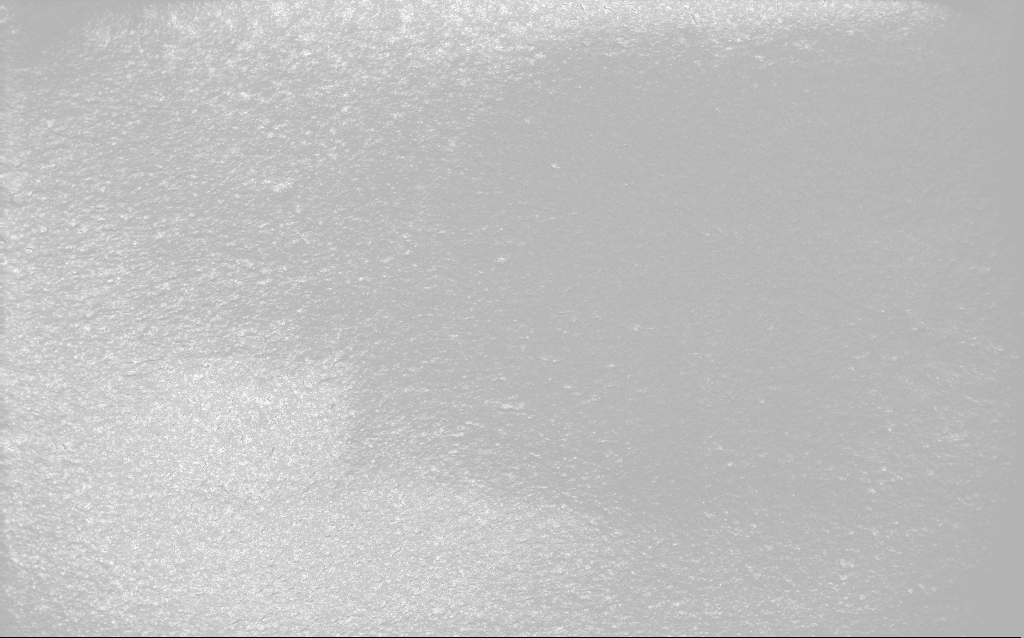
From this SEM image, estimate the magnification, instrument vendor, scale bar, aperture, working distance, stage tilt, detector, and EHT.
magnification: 0.122 K X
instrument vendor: Zeiss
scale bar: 100000 nm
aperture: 30 µm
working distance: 2.7 mm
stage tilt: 0°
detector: InLens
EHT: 10 kV